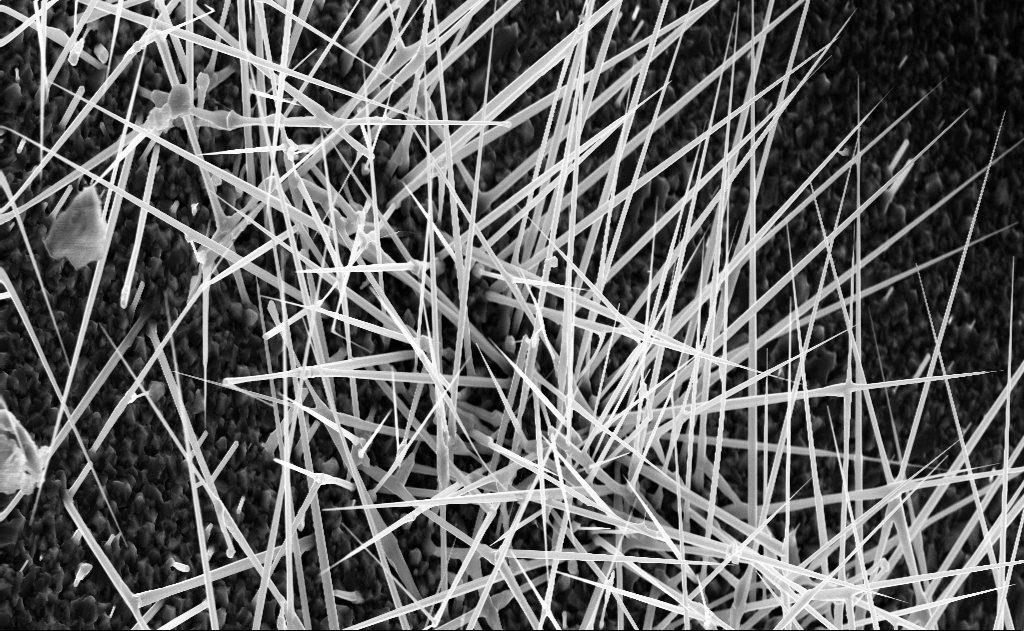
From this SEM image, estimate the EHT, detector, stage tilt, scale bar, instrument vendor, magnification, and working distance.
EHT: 10 kV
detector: InLens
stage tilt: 0°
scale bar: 2000 nm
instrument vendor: Zeiss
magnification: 10 K X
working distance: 4 mm